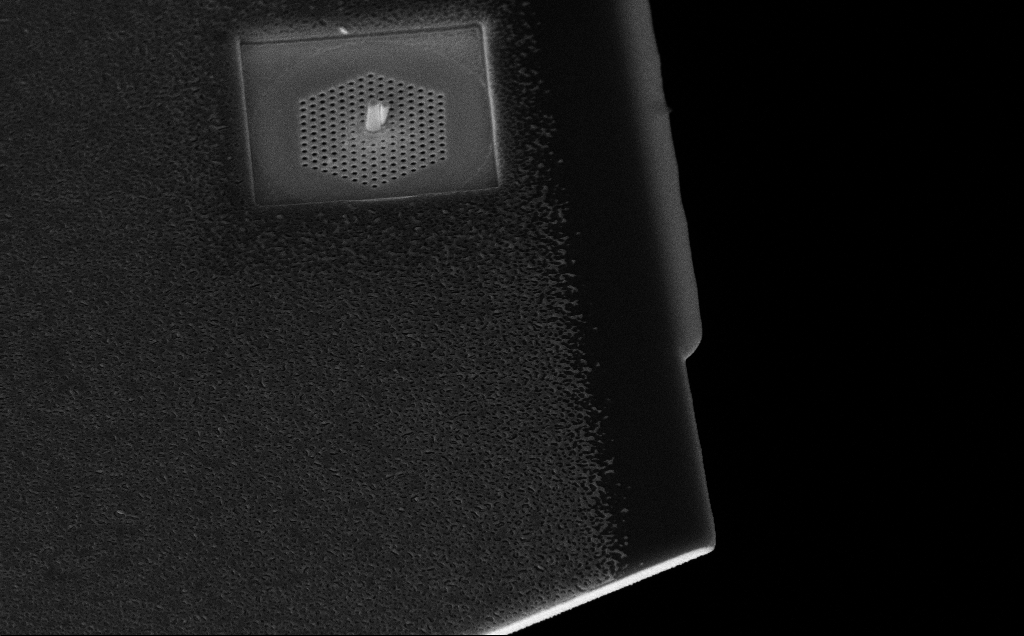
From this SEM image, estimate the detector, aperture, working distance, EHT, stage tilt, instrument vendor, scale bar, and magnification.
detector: InLens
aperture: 30 µm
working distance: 11 mm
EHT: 10 kV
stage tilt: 45°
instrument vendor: Zeiss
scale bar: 1000 nm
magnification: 14.46 K X